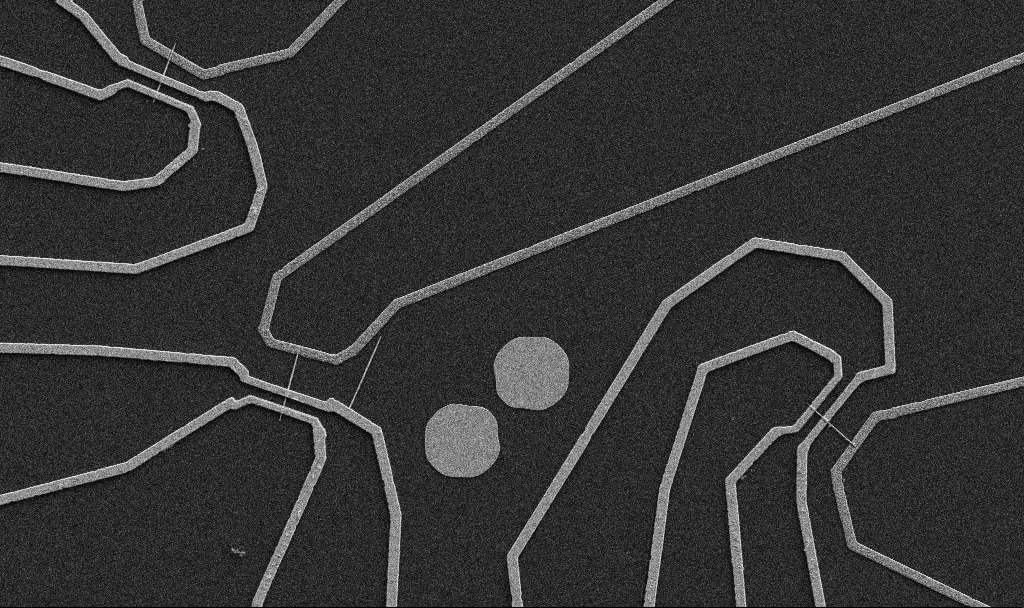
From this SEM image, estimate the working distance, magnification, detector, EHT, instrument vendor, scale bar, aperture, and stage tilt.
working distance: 10.7 mm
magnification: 5 K X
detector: SE2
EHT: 5 kV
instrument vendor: Zeiss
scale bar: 10000 nm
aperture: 30 µm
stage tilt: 0°